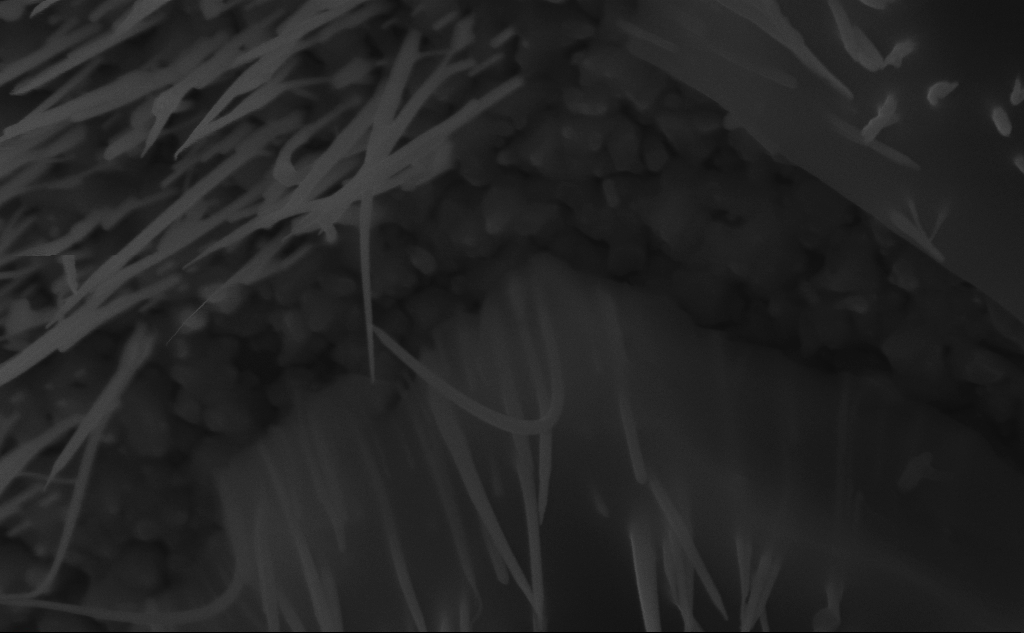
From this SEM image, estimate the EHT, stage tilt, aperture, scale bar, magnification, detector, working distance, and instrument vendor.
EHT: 10 kV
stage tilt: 45°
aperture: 30 µm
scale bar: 200 nm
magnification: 98.96 K X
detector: InLens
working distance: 6 mm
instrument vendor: Zeiss